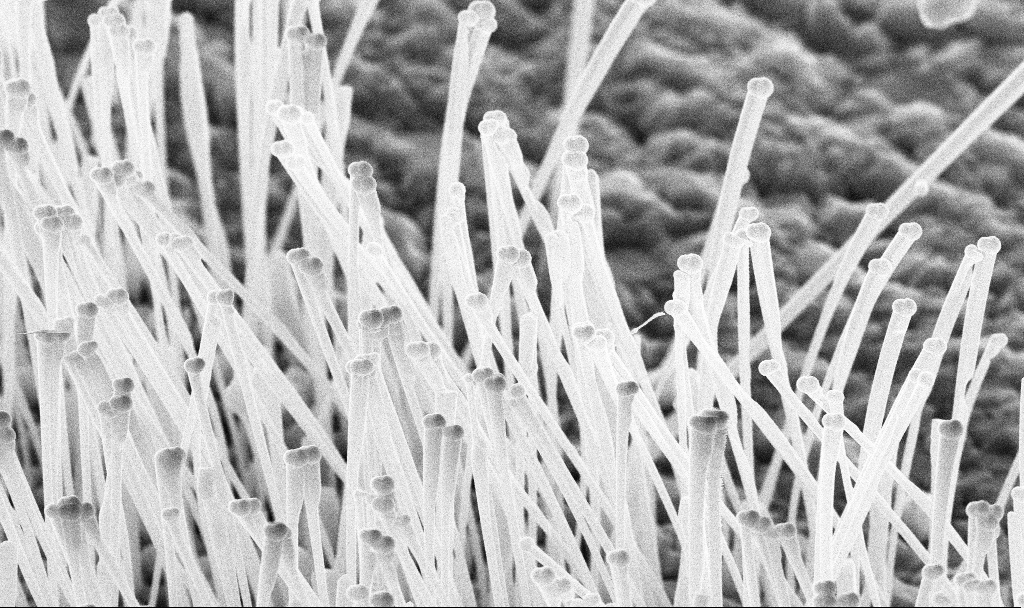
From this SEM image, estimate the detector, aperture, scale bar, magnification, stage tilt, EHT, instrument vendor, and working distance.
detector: InLens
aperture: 30 µm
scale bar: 2000 nm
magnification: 30.98 K X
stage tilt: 45°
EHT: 10 kV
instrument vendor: Zeiss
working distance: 7.1 mm